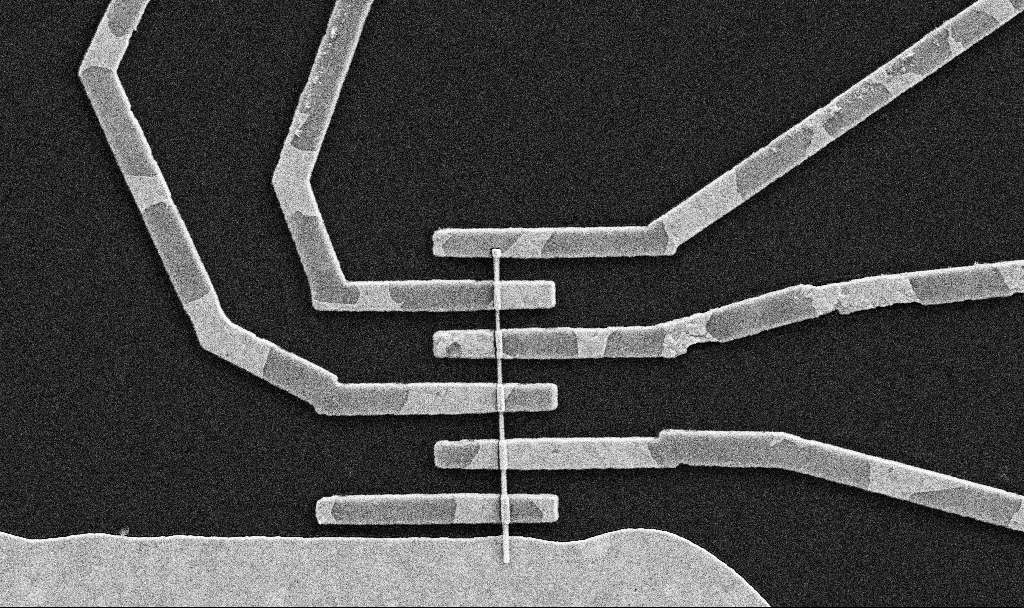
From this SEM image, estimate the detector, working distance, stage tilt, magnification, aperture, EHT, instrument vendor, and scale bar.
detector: SE2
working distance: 8.5 mm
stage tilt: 0°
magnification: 17.55 K X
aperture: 30 µm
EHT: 5 kV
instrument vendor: Zeiss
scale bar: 2000 nm